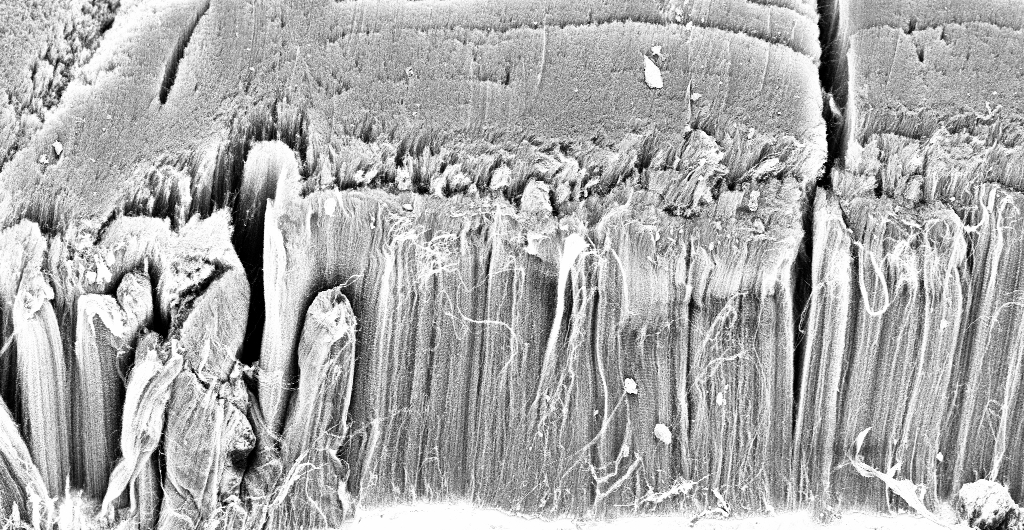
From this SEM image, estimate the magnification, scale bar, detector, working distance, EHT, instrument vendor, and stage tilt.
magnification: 1 K X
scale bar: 20000 nm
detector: InLens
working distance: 3.4 mm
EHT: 3 kV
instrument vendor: Zeiss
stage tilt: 45°